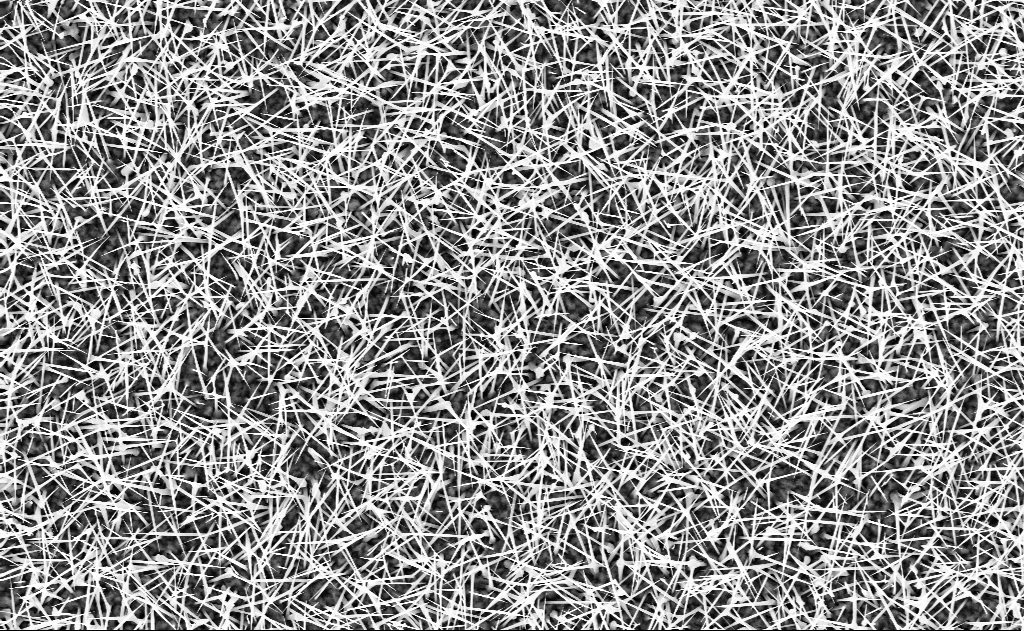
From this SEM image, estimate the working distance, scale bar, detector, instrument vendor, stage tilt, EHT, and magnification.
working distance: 9 mm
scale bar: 2000 nm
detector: InLens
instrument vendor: Zeiss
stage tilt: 0°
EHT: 10 kV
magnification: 10 K X